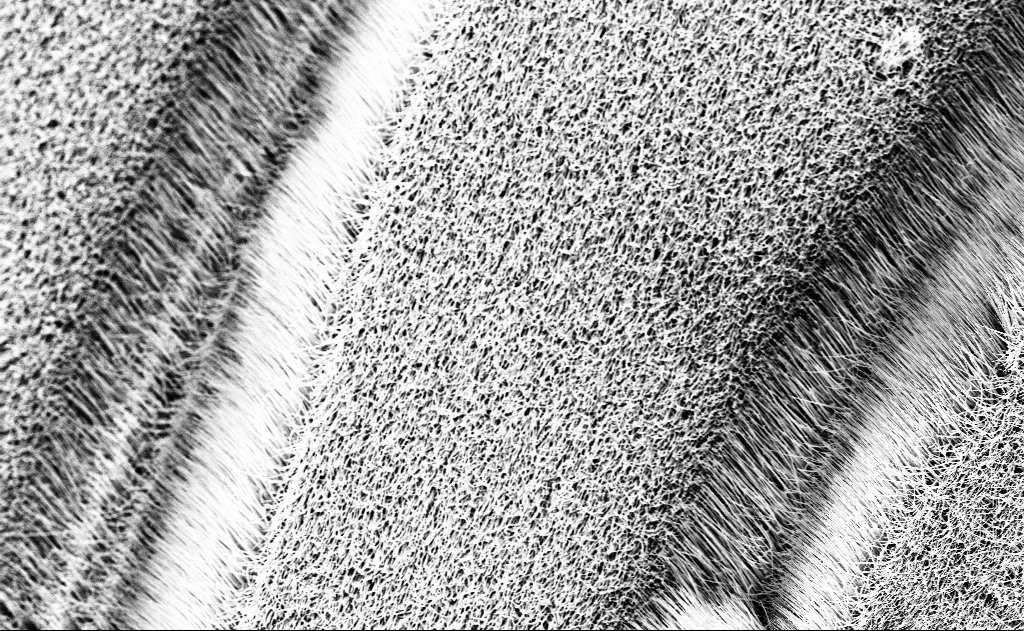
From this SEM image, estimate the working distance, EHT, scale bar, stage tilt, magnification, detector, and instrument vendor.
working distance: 12 mm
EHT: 10 kV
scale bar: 10000 nm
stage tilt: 0°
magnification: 5 K X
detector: InLens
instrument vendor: Zeiss